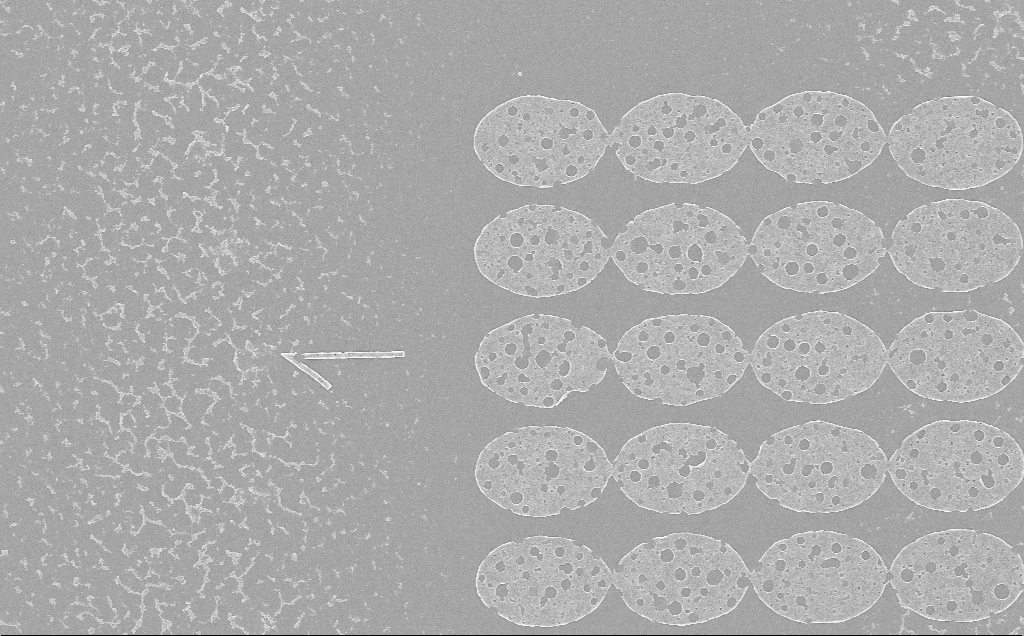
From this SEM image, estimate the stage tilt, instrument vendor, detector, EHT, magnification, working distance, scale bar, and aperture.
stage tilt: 0°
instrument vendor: Zeiss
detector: InLens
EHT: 5 kV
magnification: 5.1 K X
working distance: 6 mm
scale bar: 10000 nm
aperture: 30 µm